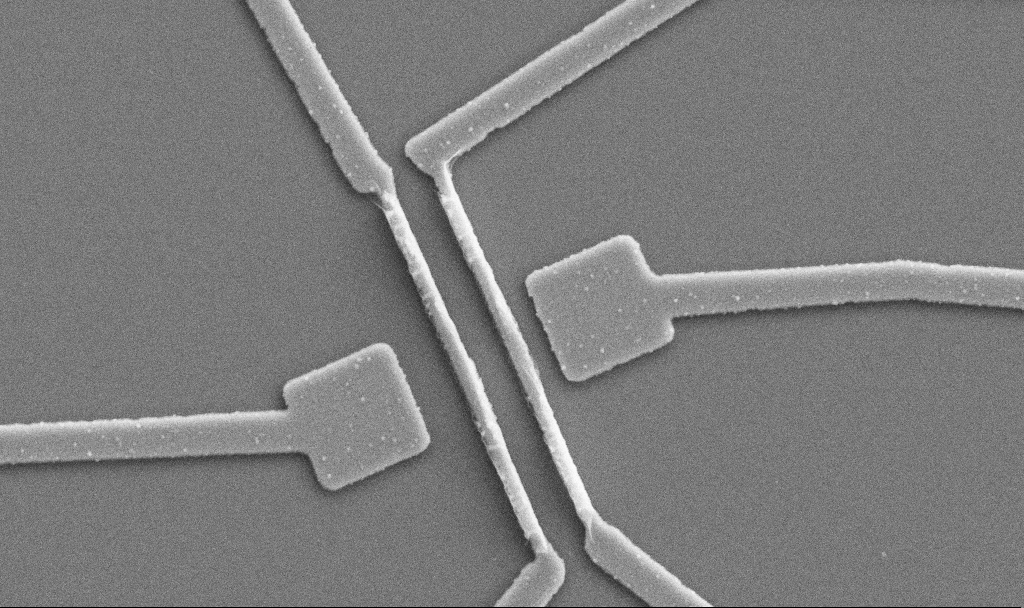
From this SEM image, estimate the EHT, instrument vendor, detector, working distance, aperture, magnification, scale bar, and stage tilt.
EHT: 5 kV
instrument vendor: Zeiss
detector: SE2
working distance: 10.7 mm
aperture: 30 µm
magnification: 20 K X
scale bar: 1000 nm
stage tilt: -0°